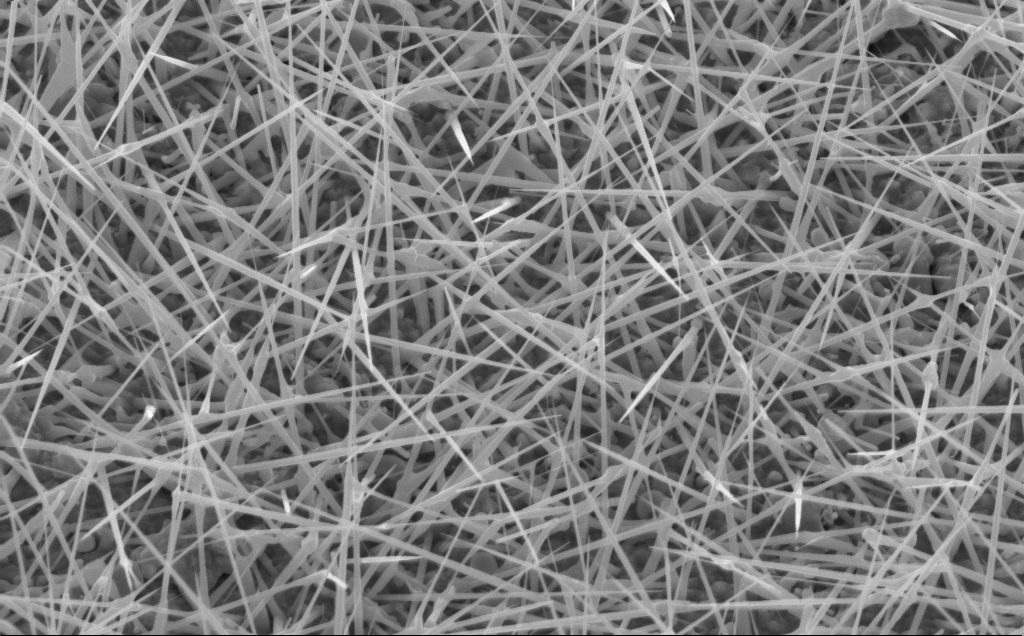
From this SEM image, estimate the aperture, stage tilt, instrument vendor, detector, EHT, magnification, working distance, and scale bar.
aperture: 30 µm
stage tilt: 30°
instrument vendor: Zeiss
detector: InLens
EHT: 10 kV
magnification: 20 K X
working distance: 5 mm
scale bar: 2000 nm